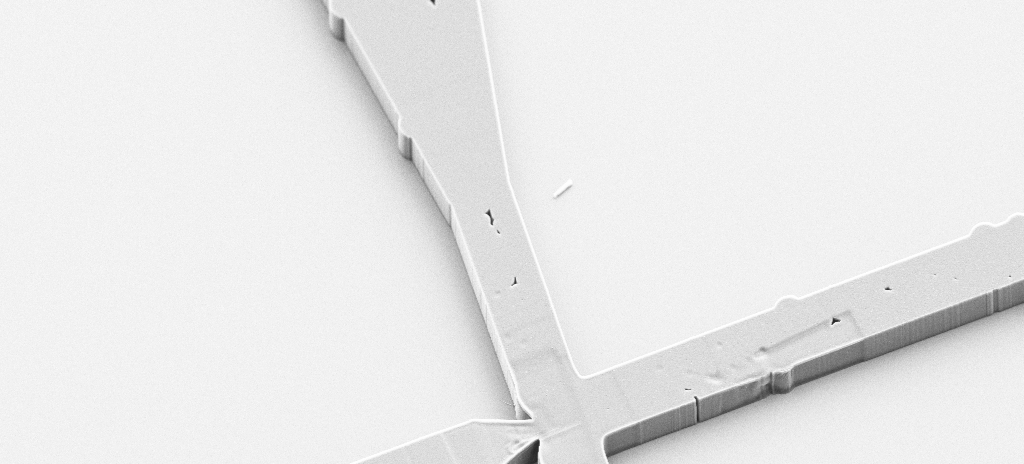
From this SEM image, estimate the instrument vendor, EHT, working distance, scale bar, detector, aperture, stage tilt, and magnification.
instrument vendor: Zeiss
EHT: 5 kV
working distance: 9 mm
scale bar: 20000 nm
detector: SE2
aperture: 30 µm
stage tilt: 45°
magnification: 2.25 K X